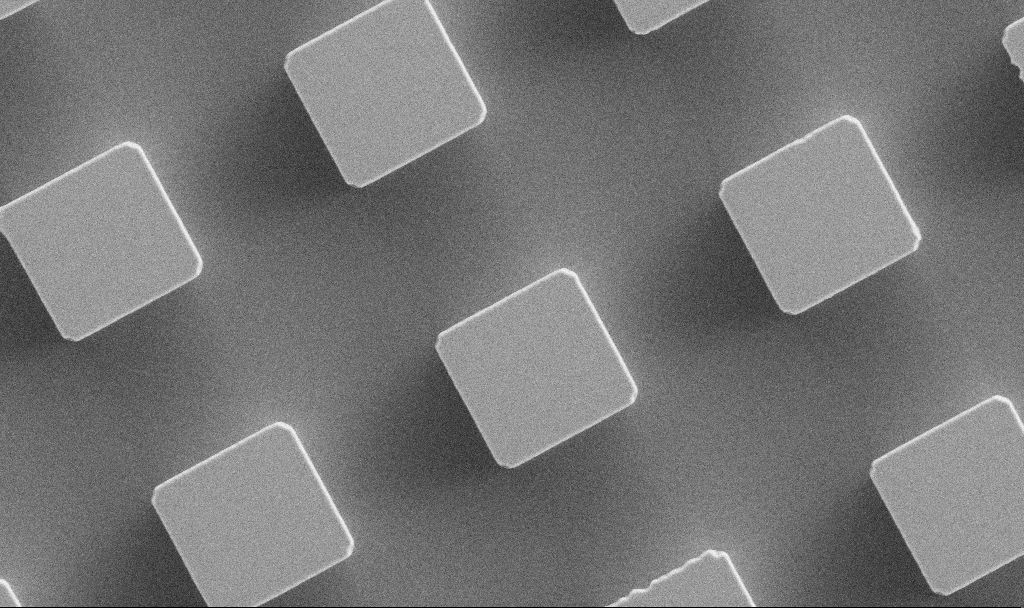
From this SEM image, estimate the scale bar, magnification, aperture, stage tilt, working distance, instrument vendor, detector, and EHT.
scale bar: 10000 nm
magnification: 5.94 K X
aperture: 30 µm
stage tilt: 0°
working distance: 5.6 mm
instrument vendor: Zeiss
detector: SE2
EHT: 5 kV